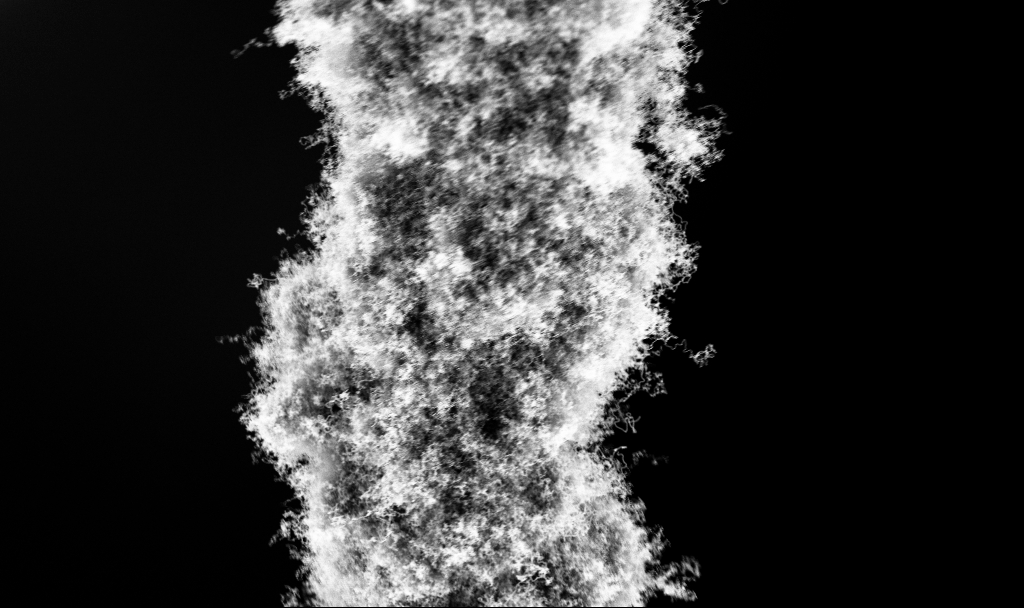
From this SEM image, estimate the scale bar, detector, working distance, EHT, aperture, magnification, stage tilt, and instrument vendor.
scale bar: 2000 nm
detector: InLens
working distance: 2.7 mm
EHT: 3 kV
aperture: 30 µm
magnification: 10 K X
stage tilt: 0°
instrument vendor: Zeiss